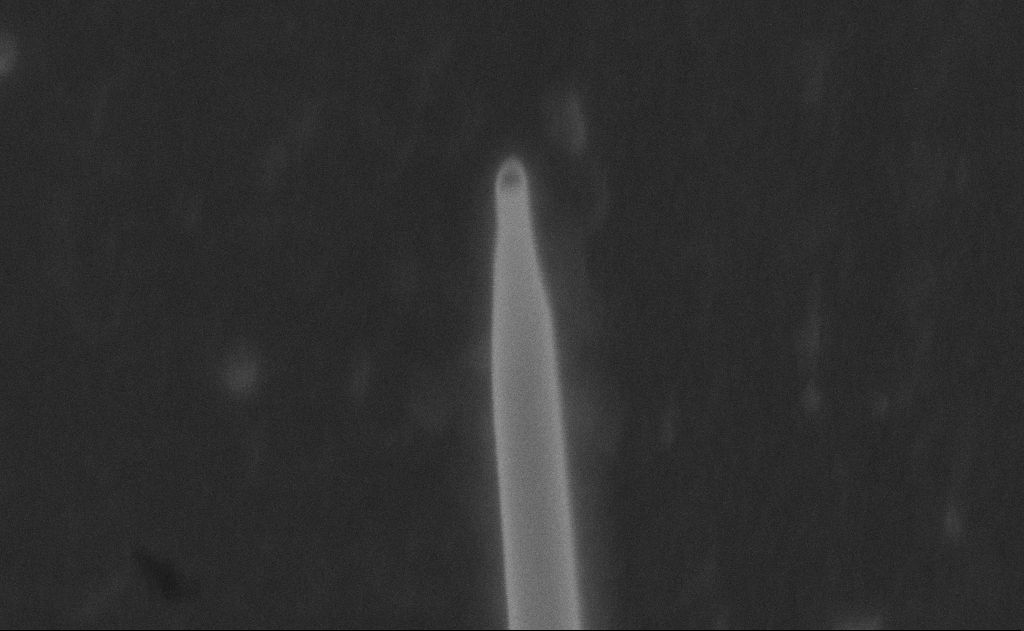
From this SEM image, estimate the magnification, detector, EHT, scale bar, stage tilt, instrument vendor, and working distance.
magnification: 265.18 K X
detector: SE2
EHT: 20 kV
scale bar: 200 nm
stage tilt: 0°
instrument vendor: Zeiss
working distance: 9 mm